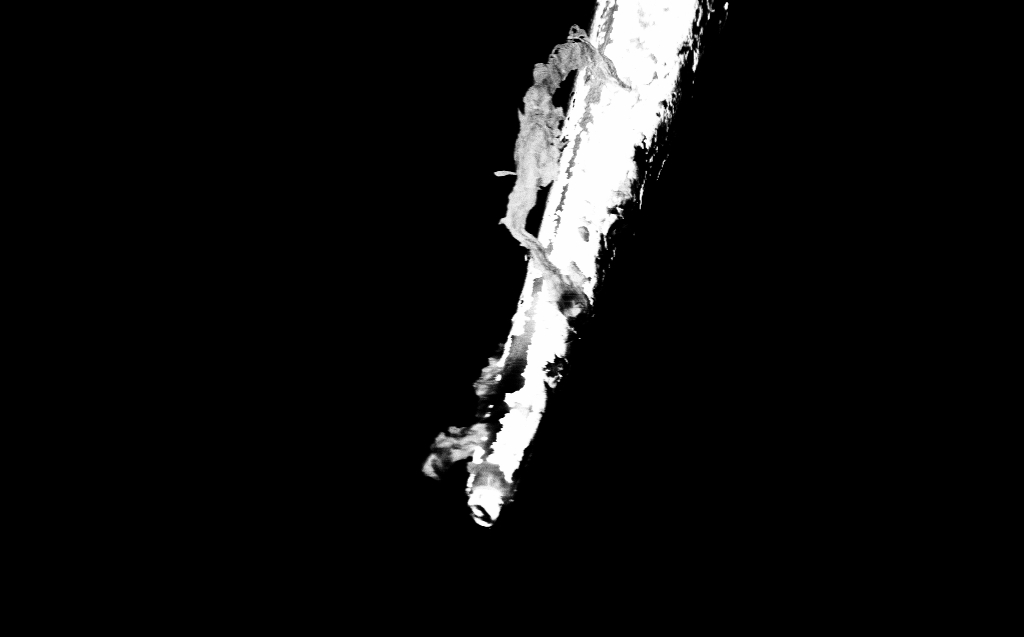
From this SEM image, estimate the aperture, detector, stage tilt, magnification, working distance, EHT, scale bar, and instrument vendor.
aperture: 30 µm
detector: InLens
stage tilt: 45°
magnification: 4.87 K X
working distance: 4 mm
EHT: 1 kV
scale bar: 10000 nm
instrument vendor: Zeiss